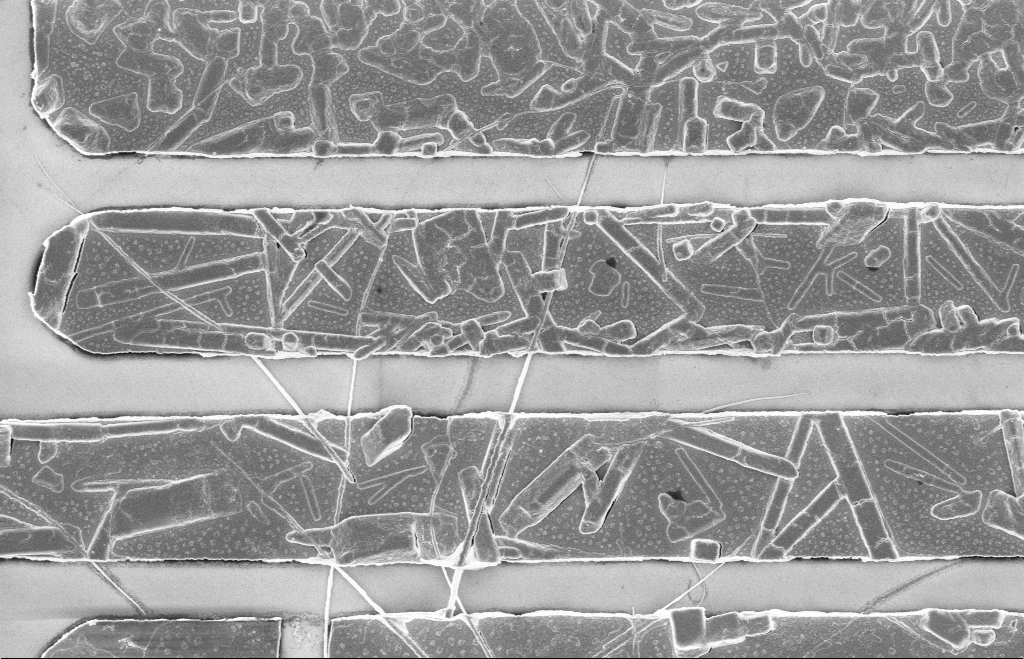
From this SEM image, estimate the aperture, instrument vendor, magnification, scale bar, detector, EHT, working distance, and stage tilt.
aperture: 20 µm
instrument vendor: Zeiss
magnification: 12.59 K X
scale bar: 2000 nm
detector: InLens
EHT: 5 kV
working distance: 8 mm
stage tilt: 0°